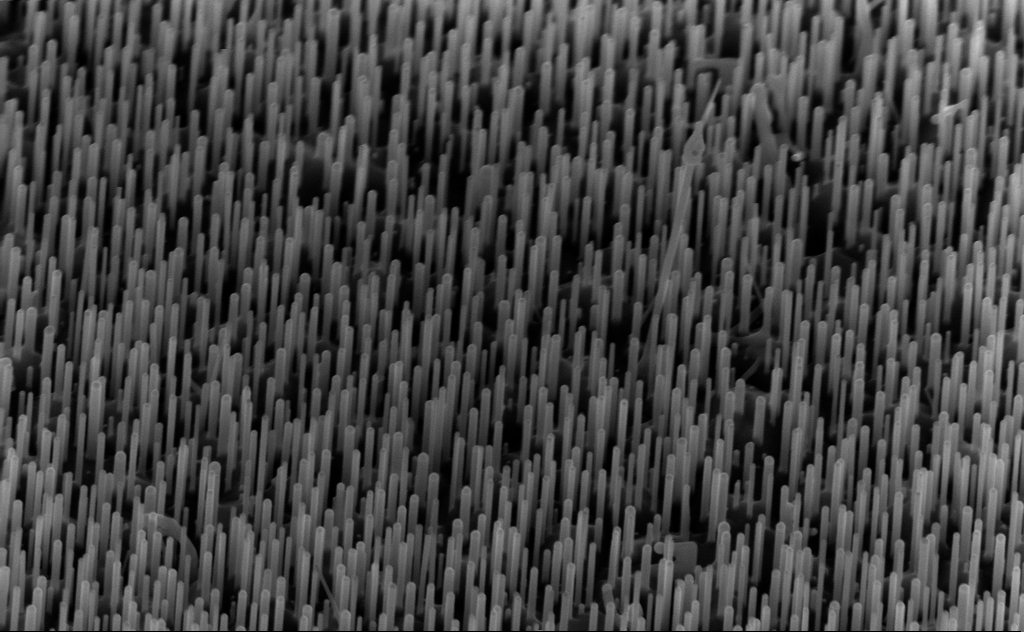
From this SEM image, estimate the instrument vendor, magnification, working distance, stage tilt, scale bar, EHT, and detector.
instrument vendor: Zeiss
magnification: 60 K X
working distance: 6 mm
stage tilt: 45°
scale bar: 1000 nm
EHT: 10 kV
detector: InLens